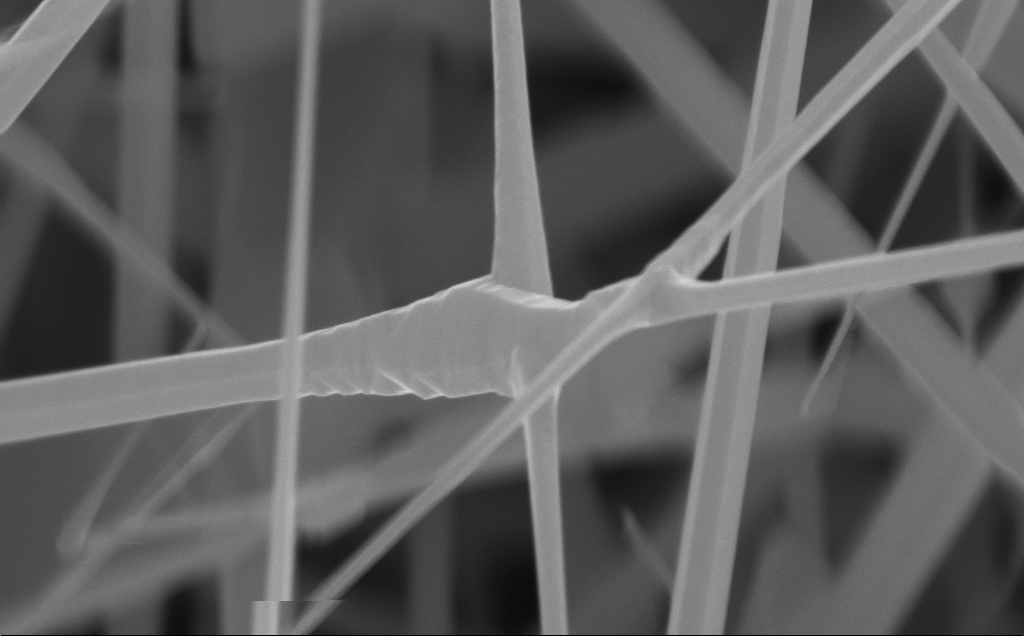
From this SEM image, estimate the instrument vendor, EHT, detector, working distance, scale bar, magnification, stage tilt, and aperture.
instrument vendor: Zeiss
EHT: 10 kV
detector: InLens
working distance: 4 mm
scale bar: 200 nm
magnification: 108.91 K X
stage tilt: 0°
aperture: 30 µm